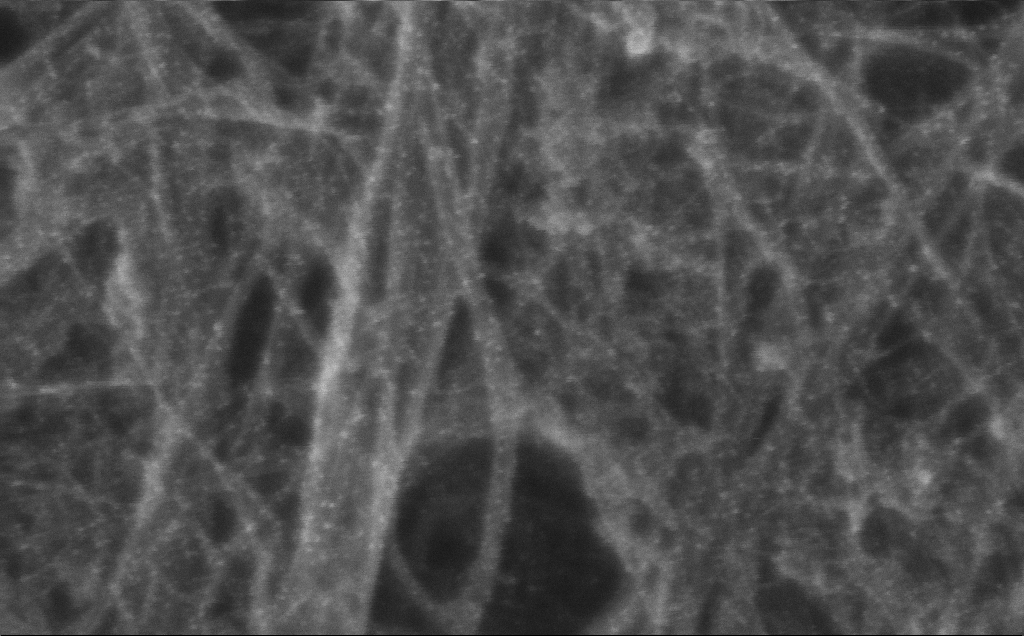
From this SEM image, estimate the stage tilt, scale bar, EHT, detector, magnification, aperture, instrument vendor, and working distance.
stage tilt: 0°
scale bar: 100 nm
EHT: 10 kV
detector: InLens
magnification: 404.09 K X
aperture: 30 µm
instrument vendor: Zeiss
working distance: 3 mm